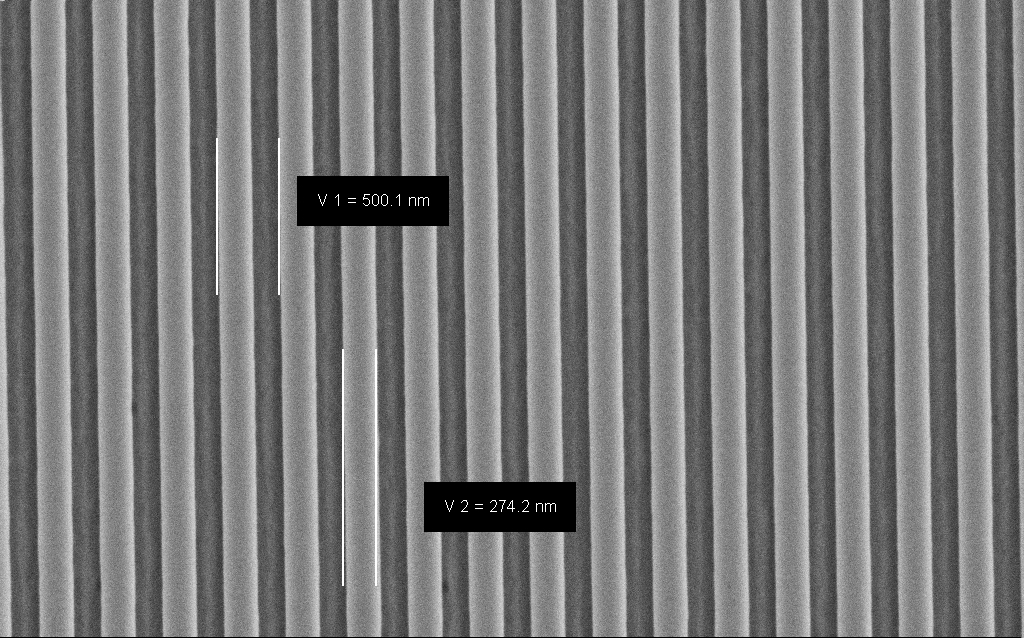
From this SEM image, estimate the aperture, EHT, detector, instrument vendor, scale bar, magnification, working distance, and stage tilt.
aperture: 30 µm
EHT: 3 kV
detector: SE2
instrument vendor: Zeiss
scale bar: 1000 nm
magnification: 45.52 K X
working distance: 5 mm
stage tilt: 0°